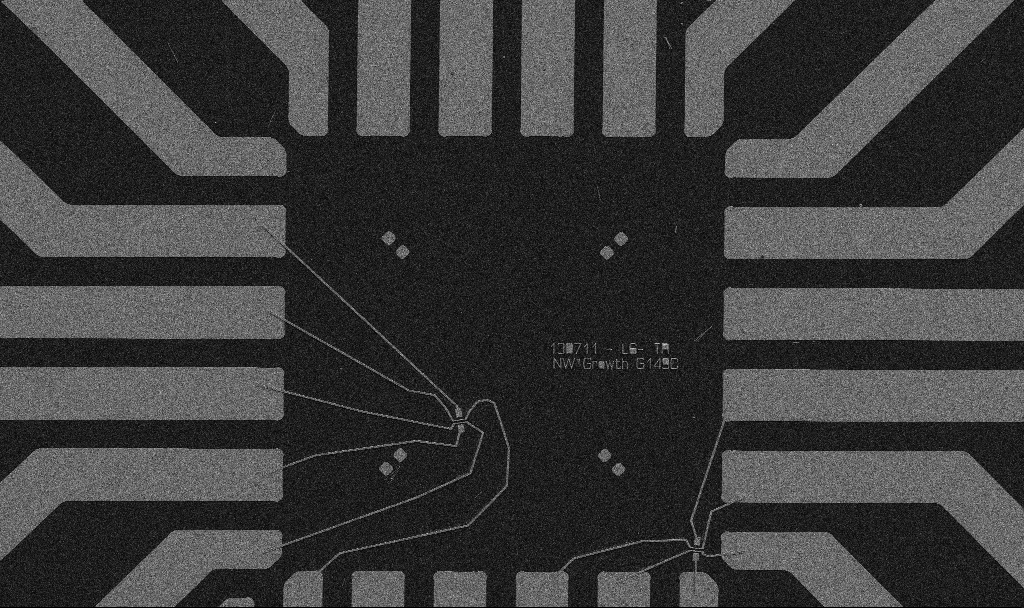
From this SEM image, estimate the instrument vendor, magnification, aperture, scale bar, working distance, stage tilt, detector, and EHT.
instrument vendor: Zeiss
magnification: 1 K X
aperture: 30 µm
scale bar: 20000 nm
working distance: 10.7 mm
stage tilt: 0°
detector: SE2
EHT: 5 kV